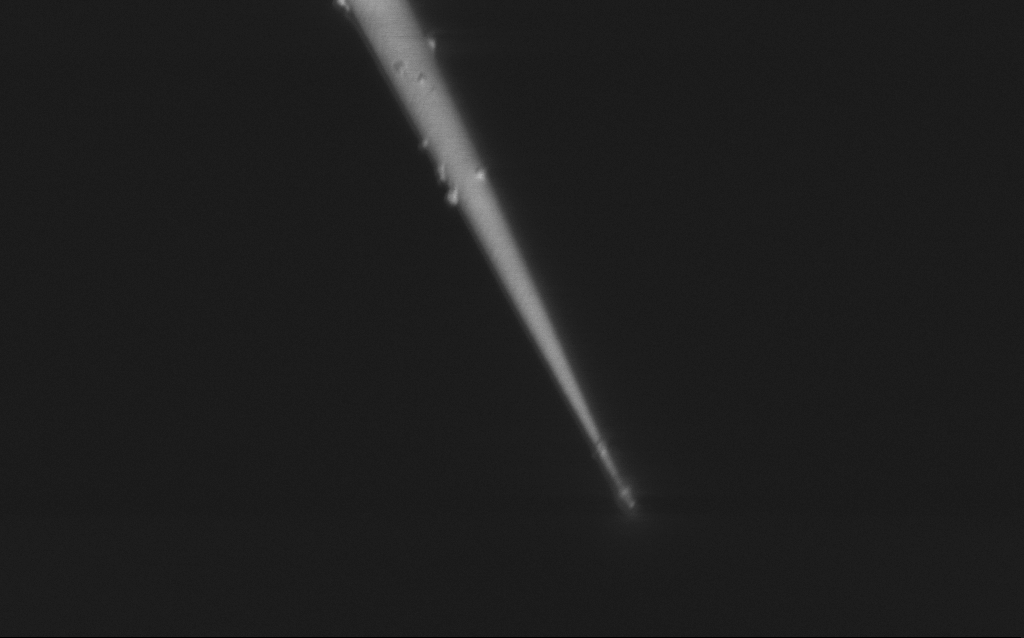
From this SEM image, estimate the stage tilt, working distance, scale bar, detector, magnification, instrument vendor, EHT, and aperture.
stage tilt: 45°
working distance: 7 mm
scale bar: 1000 nm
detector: InLens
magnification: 46.01 K X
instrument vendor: Zeiss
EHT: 1 kV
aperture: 30 µm